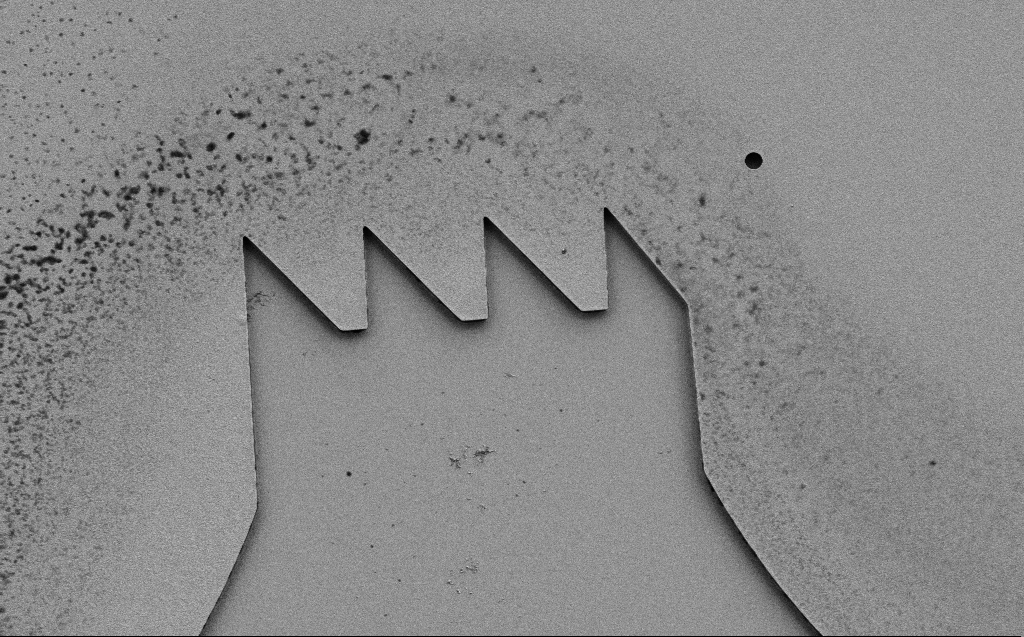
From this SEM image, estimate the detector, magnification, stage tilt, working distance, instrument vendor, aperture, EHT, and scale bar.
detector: SE2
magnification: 3.86 K X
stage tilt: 0°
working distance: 9 mm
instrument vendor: Zeiss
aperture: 30 µm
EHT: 3 kV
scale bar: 10000 nm